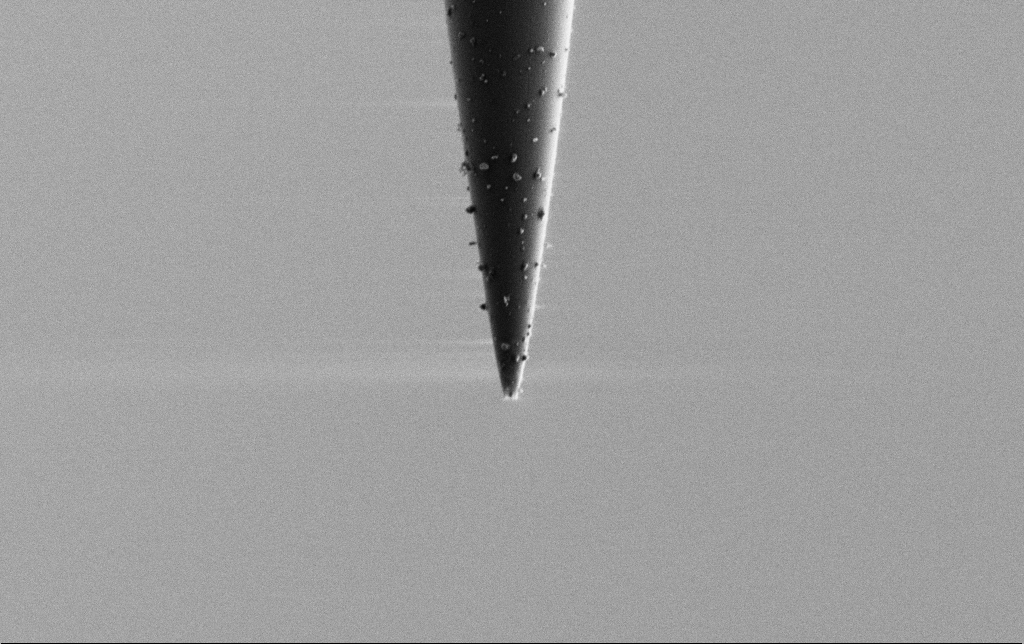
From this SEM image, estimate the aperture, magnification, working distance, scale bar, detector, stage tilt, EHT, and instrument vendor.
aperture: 30 µm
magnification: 10 K X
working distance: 6.5 mm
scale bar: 2000 nm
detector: SE2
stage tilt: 0°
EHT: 2 kV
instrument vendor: Zeiss